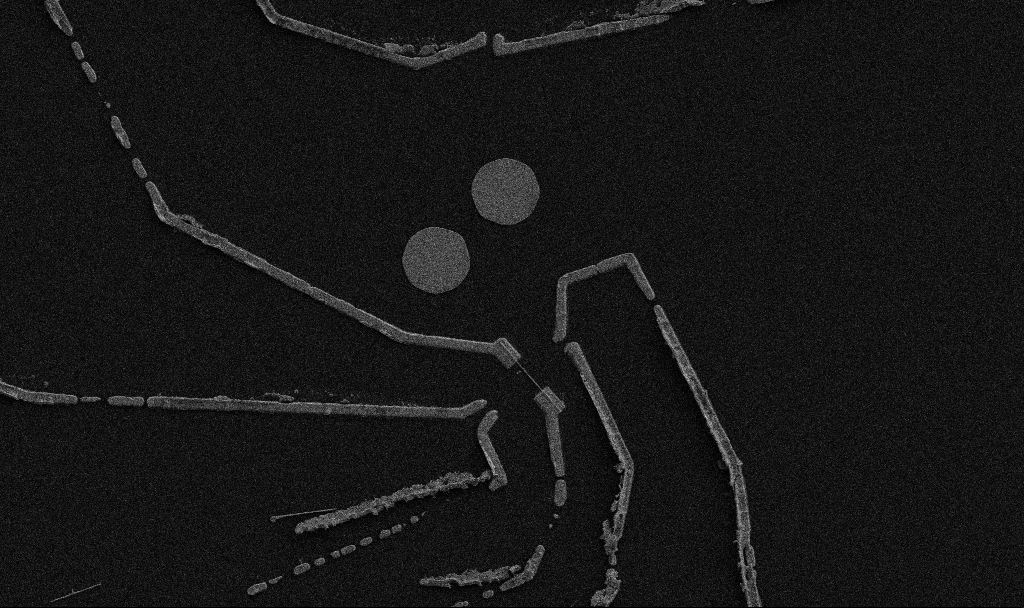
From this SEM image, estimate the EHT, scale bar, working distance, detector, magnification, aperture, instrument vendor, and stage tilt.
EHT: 5 kV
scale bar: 10000 nm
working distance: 10.7 mm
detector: SE2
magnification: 5 K X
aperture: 30 µm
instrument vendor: Zeiss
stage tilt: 0°